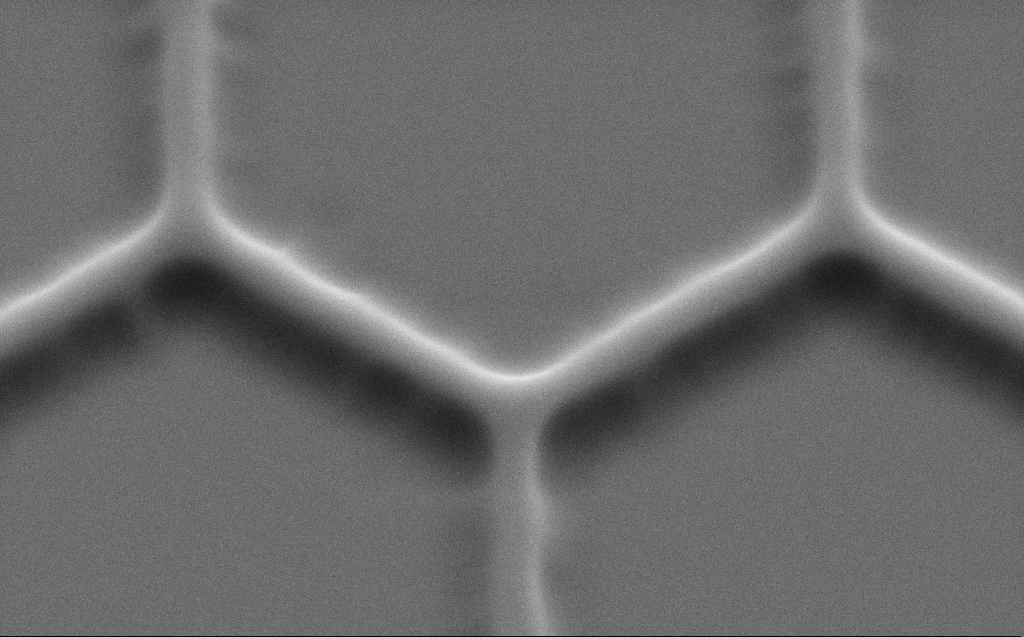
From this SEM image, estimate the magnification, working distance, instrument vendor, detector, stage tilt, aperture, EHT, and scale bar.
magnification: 38.69 K X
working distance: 6 mm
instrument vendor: Zeiss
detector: SE2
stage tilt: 45°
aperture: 30 µm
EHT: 5 kV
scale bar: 1000 nm